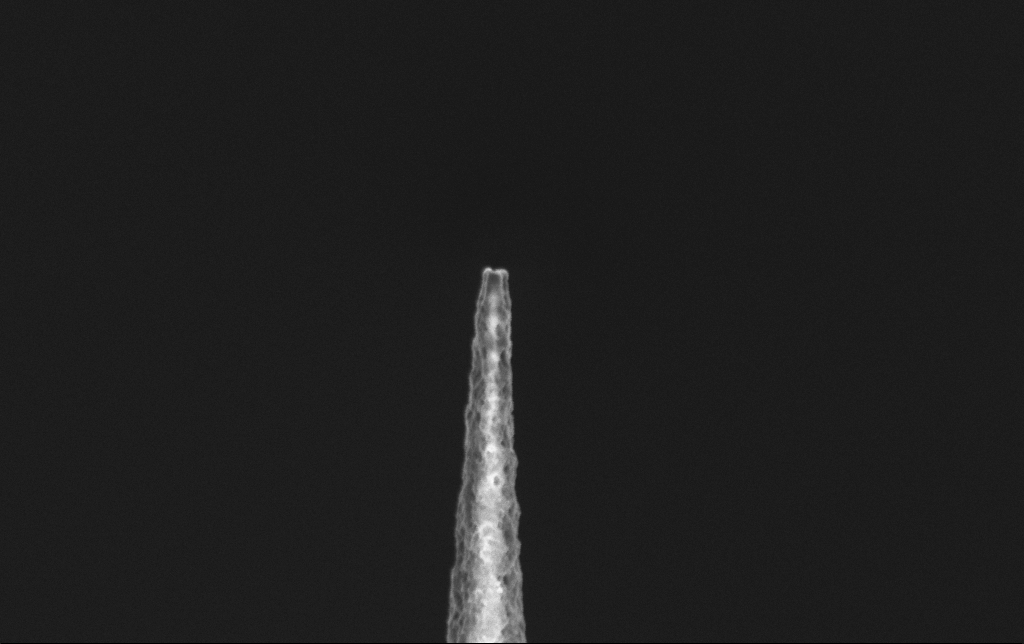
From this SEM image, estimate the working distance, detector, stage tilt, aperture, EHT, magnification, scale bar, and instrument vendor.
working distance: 6.5 mm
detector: InLens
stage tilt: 0°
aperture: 30 µm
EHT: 2 kV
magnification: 50 K X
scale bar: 1000 nm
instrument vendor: Zeiss